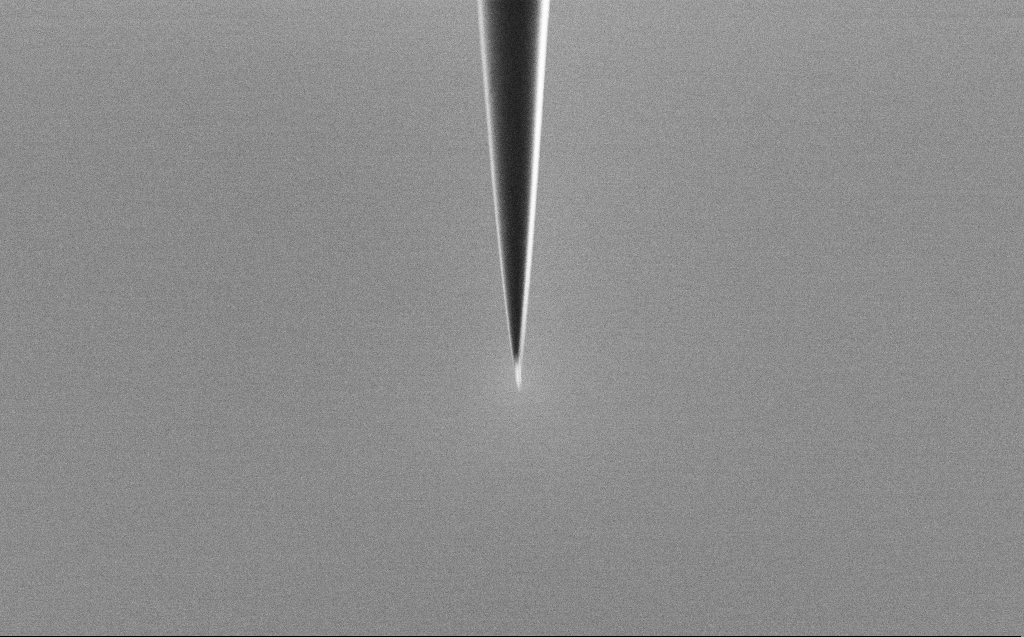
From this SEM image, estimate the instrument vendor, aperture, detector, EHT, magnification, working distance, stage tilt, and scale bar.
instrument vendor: Zeiss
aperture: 30 µm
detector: SE2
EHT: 2 kV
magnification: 10 K X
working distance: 6 mm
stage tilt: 45°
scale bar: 2000 nm